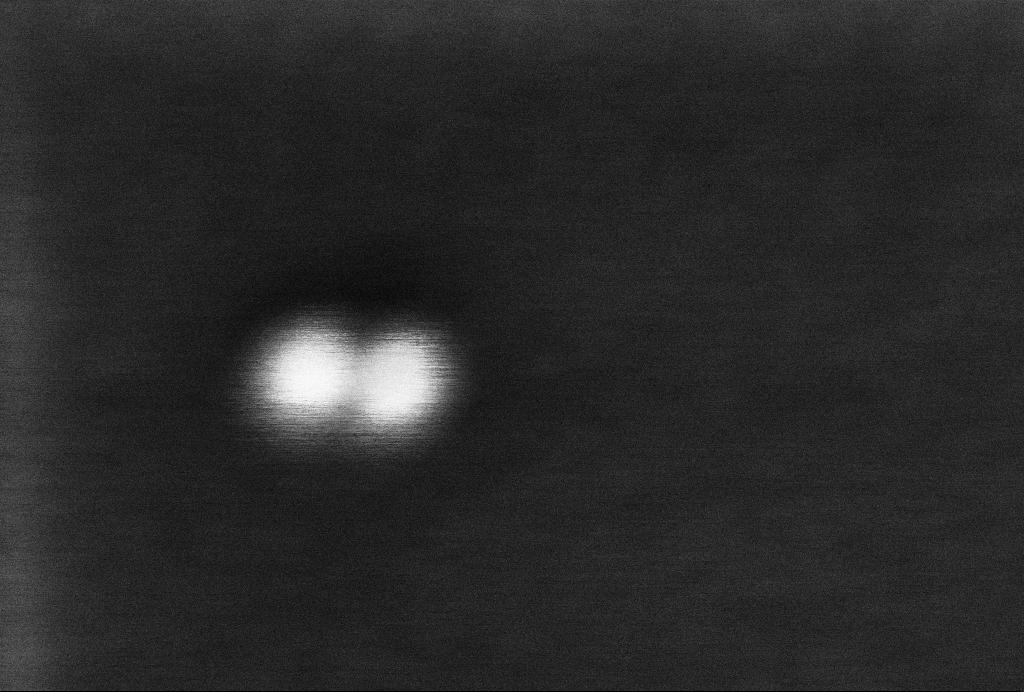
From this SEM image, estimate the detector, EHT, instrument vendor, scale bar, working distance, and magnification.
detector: InLens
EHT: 2 kV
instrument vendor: Zeiss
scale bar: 20 nm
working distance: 3.3 mm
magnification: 1375.8 K X